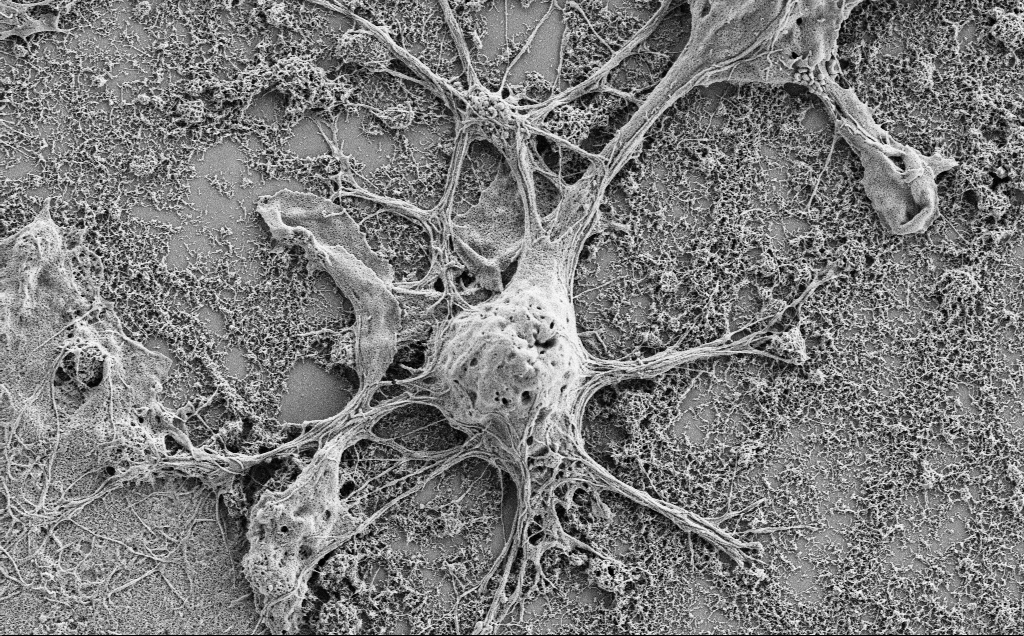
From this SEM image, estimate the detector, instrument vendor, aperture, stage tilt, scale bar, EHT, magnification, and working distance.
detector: SE2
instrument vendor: Zeiss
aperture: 30 µm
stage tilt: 0°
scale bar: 2000 nm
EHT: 2 kV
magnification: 7.5 K X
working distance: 7 mm